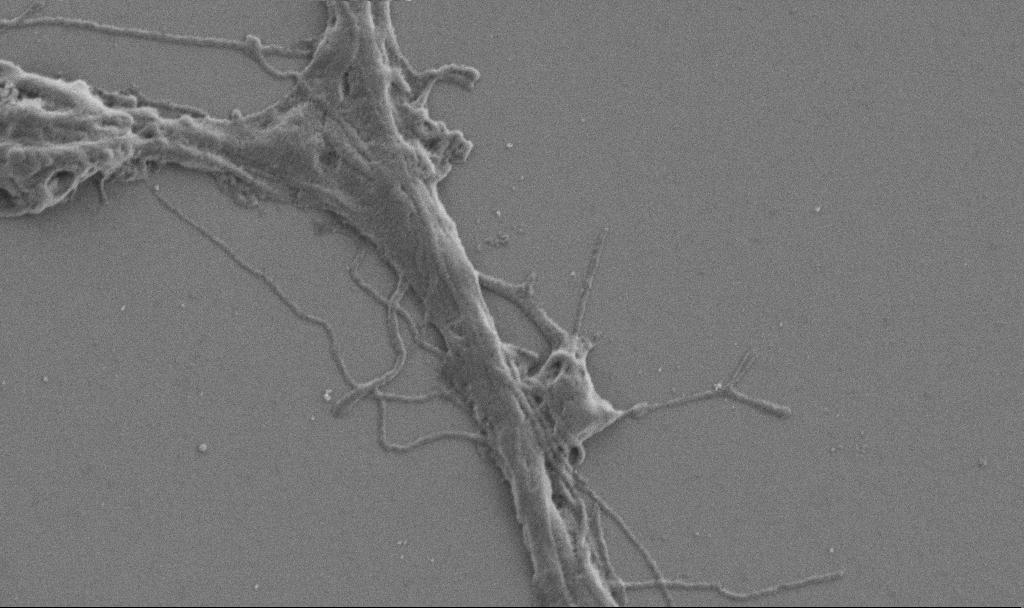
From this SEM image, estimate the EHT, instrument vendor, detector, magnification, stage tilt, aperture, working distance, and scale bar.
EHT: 1 kV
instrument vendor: Zeiss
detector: SE2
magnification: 20 K X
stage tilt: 0°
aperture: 30 µm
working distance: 6.9 mm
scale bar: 2000 nm